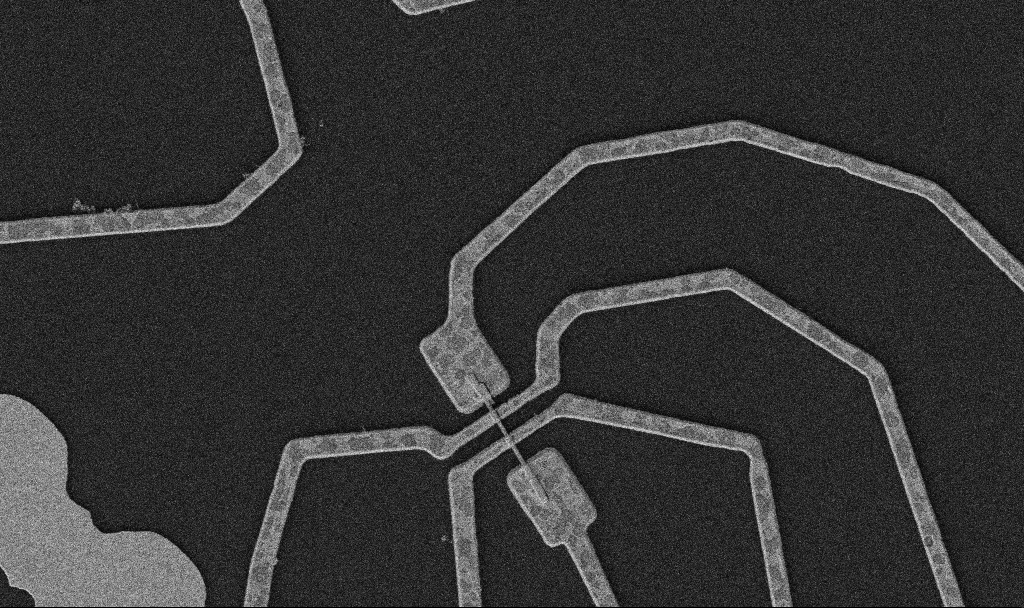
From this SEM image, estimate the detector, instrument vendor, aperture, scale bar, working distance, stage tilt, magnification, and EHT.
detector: SE2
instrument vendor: Zeiss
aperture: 30 µm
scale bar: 2000 nm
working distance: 10.7 mm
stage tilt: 0°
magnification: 10 K X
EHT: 5 kV